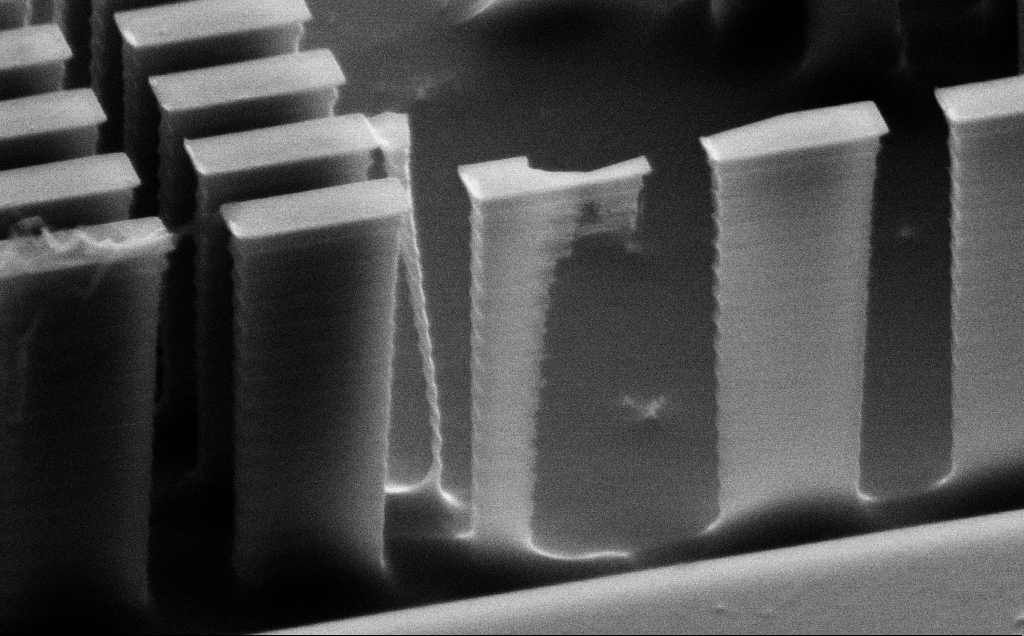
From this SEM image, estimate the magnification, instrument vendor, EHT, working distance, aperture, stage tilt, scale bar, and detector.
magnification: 22.9 K X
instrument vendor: Zeiss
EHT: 10 kV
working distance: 8 mm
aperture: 30 µm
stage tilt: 62°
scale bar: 2000 nm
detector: SE2